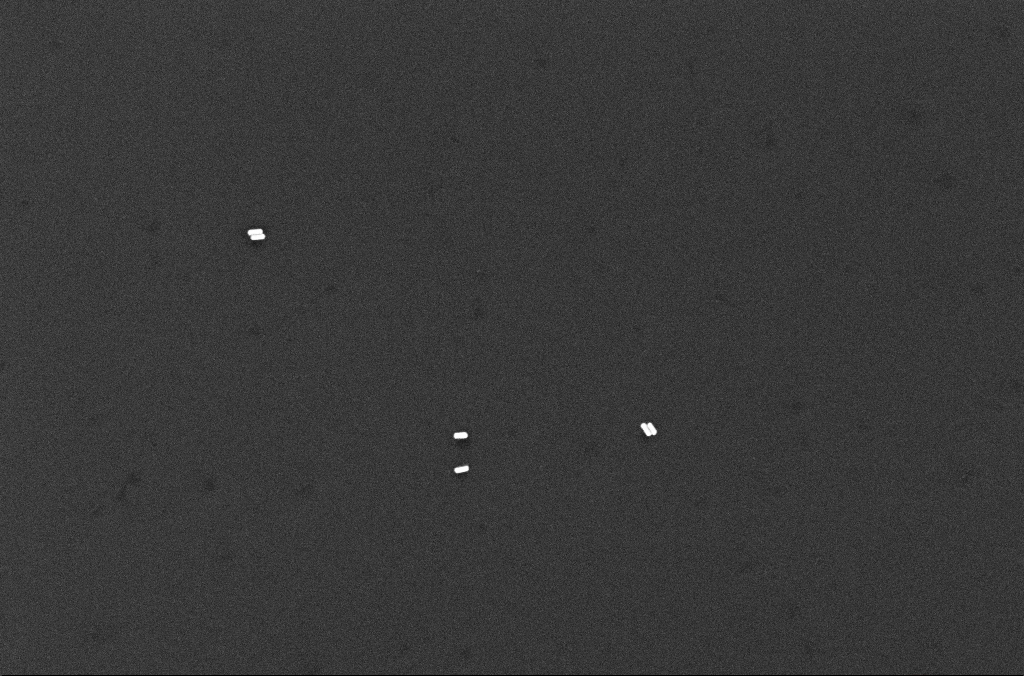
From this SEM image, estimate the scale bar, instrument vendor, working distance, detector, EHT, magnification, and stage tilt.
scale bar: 200 nm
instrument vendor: Zeiss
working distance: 2.5 mm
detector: InLens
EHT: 10 kV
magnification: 71.61 K X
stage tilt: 0°